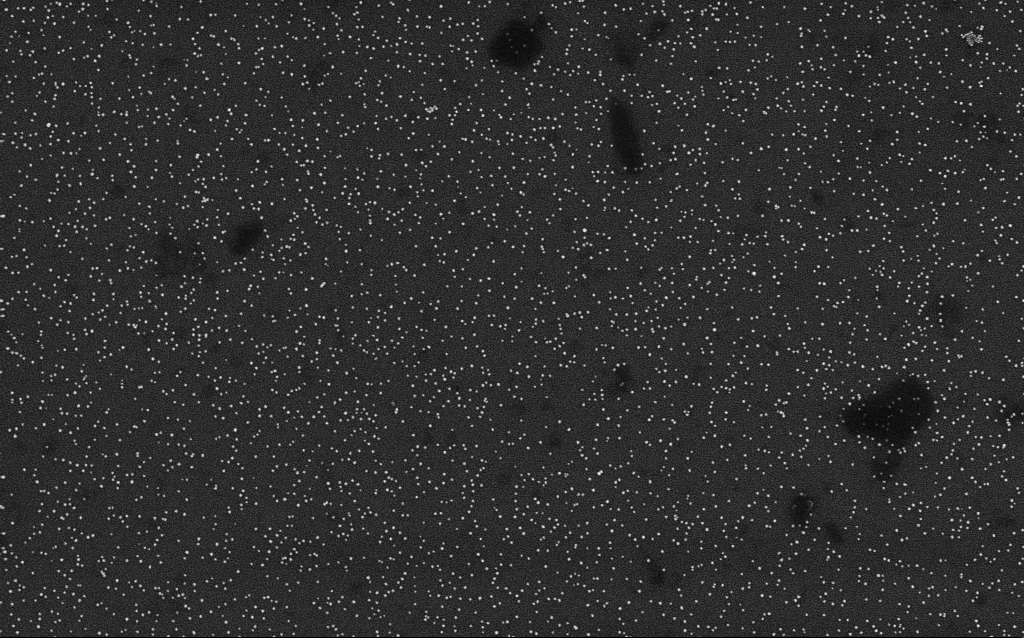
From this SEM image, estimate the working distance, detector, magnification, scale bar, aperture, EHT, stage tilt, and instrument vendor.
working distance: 2.1 mm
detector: InLens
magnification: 50 K X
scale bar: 1000 nm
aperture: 30 µm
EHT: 4 kV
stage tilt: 0°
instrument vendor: Zeiss